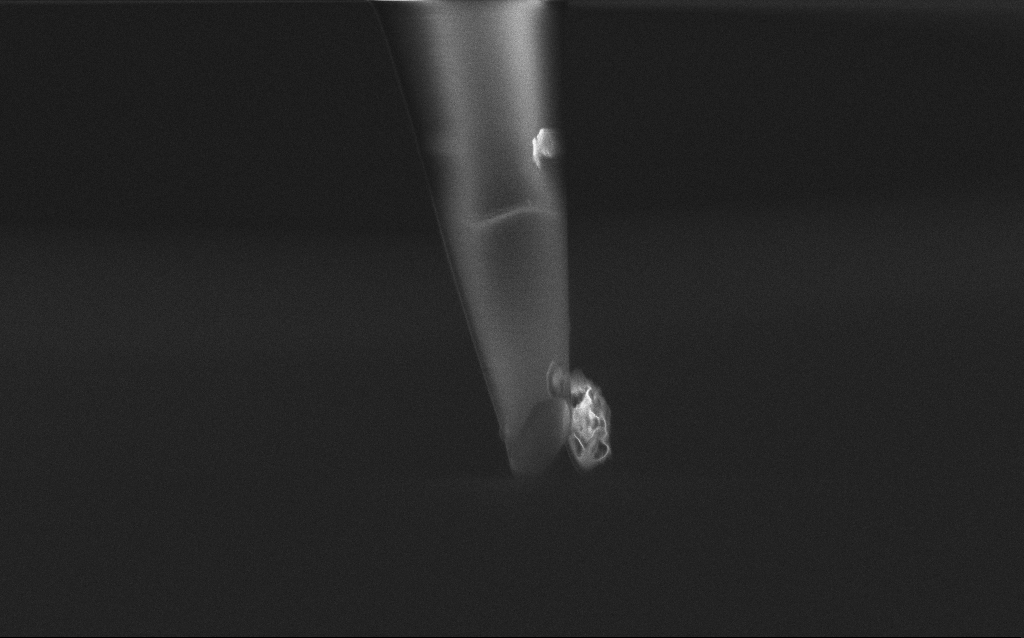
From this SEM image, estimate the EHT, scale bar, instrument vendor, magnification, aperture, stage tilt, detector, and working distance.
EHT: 2 kV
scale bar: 1000 nm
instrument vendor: Zeiss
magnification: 35.78 K X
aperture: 30 µm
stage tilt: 45°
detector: InLens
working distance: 5 mm